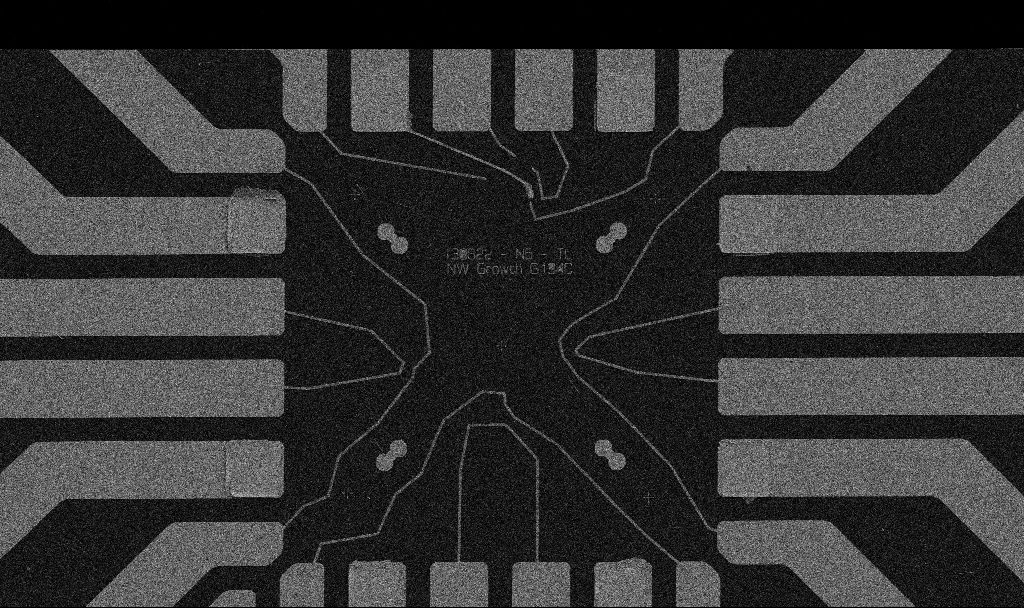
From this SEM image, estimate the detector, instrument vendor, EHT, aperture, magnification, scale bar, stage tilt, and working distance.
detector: SE2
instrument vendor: Zeiss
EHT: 5 kV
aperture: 30 µm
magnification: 1 K X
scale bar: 20000 nm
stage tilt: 0°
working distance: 10.7 mm